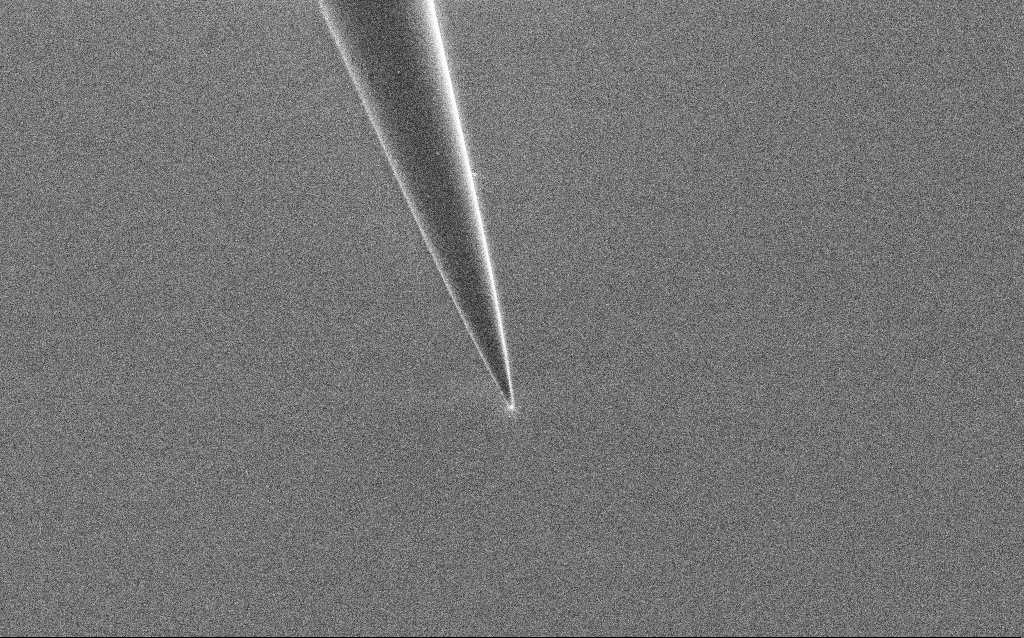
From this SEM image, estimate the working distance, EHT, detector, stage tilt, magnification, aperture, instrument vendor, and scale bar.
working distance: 7 mm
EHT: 1 kV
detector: SE2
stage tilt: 45°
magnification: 10 K X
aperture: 30 µm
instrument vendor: Zeiss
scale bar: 2000 nm